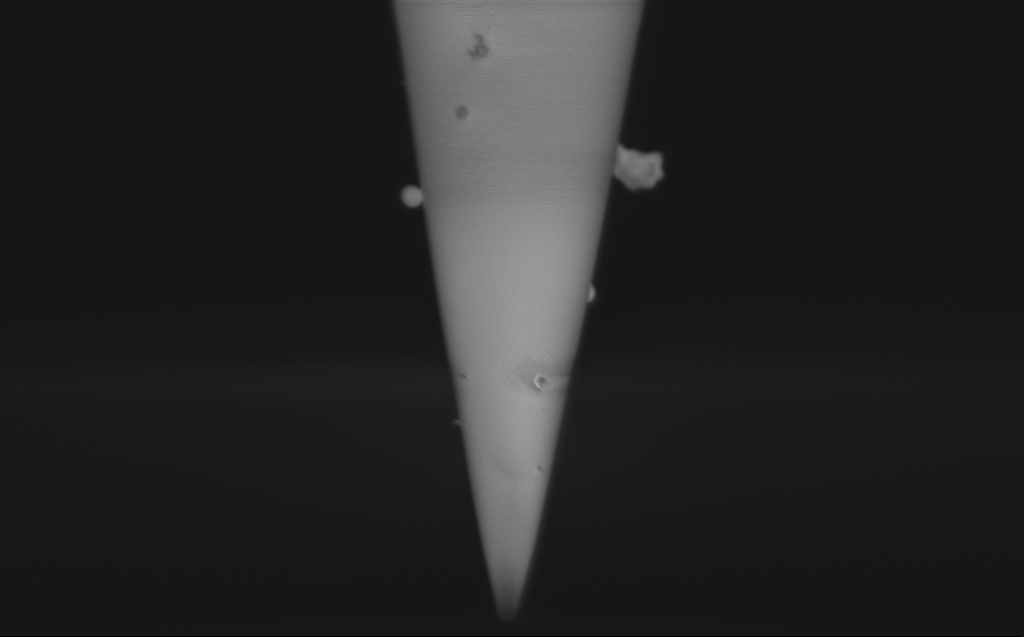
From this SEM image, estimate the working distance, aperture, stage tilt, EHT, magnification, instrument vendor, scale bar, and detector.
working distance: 3 mm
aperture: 30 µm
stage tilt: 45.1°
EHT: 1 kV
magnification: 57.53 K X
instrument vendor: Zeiss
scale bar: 1000 nm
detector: InLens